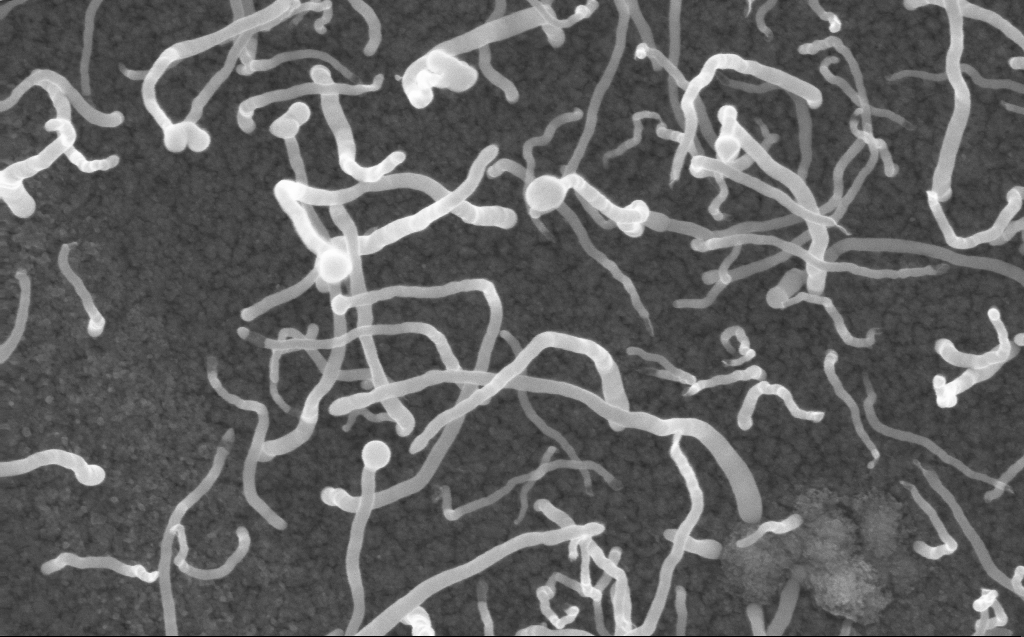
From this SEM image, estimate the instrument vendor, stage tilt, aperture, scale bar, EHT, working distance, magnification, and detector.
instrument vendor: Zeiss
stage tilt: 0°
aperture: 30 µm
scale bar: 200 nm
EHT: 10 kV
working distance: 3 mm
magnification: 100 K X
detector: InLens